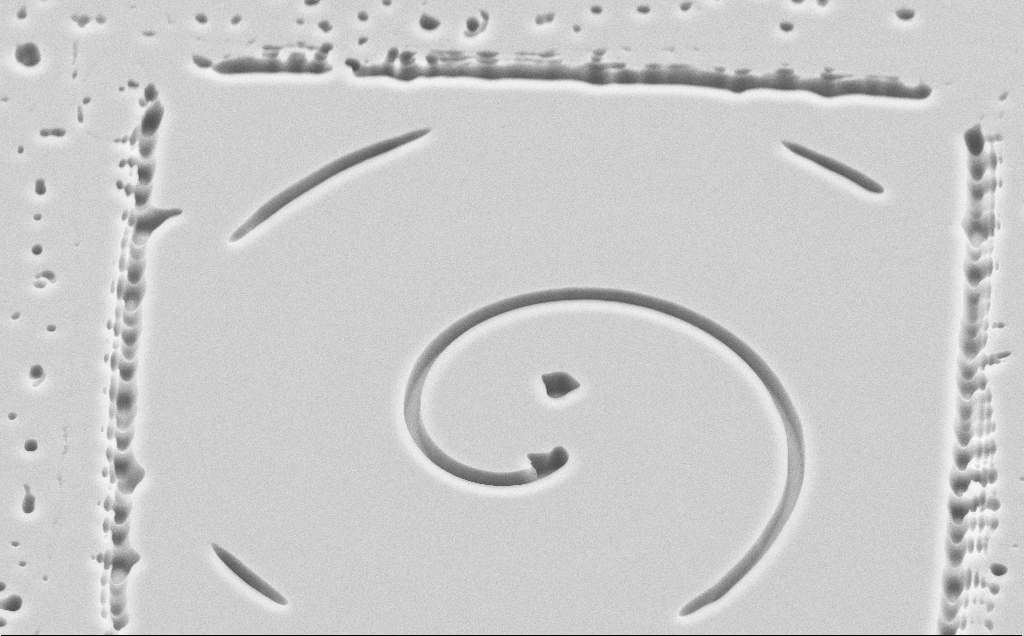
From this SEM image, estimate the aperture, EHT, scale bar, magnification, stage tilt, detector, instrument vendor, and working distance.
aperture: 30 µm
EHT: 10 kV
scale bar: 20000 nm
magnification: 3.59 K X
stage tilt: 45°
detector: SE2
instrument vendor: Zeiss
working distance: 6 mm